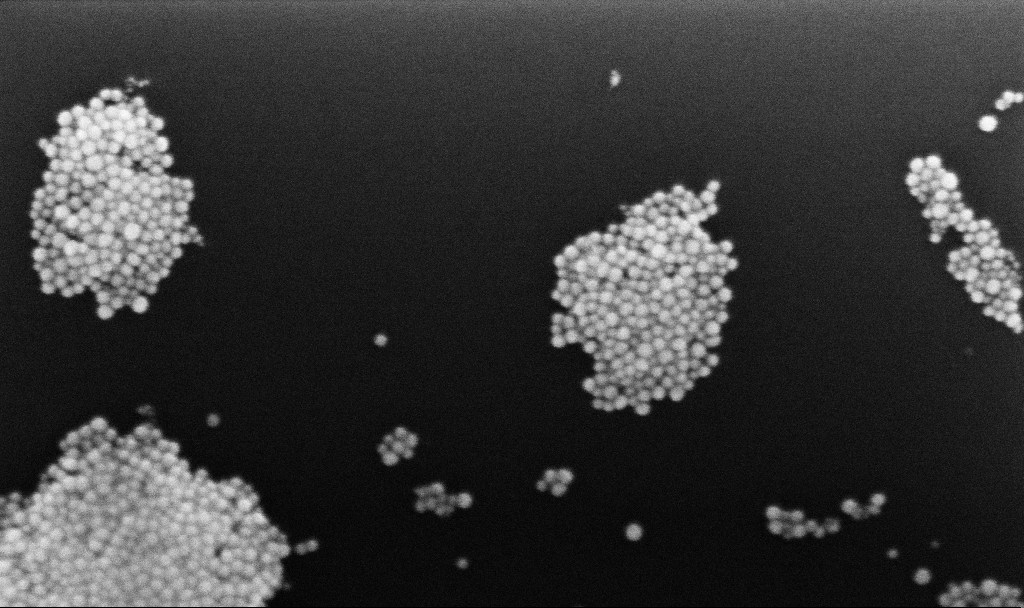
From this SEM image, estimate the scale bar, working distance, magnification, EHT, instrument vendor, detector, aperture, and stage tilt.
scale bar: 100 nm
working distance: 3.1 mm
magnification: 271.23 K X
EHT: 10 kV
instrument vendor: Zeiss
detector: InLens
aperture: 30 µm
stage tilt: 0°